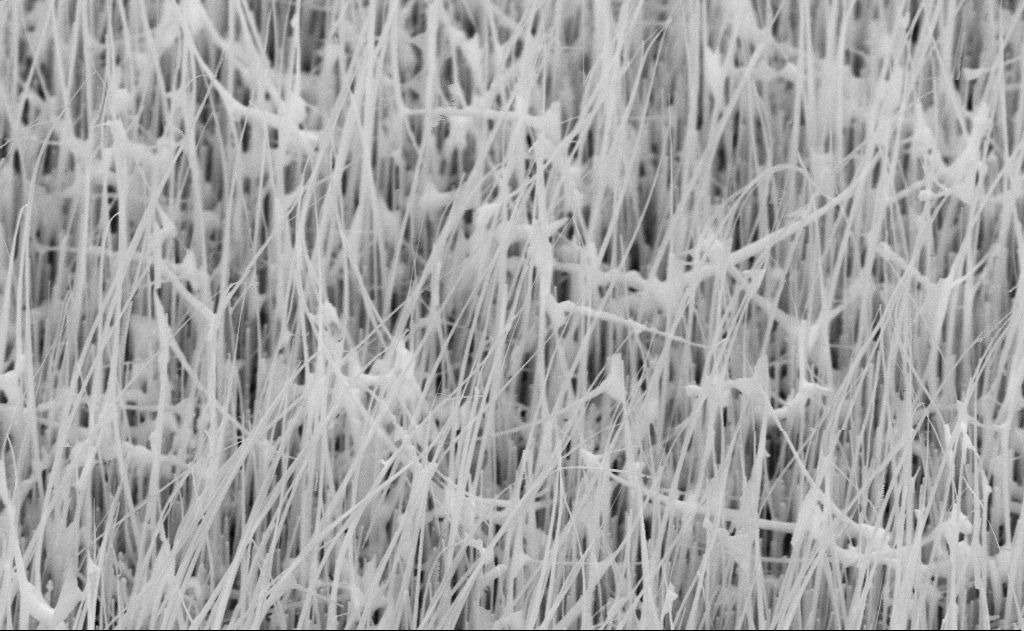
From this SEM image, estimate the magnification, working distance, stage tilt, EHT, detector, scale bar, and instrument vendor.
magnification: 40 K X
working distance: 15 mm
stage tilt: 45°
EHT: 10 kV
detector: SE2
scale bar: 1000 nm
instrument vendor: Zeiss